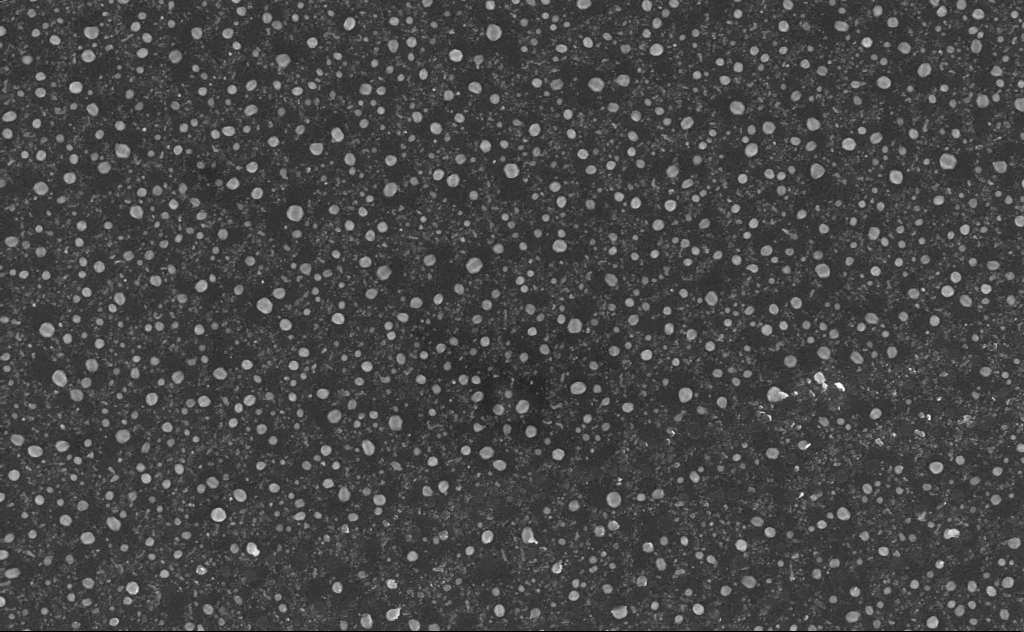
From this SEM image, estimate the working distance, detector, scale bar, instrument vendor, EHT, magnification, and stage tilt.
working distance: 4 mm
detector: InLens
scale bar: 1000 nm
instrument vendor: Zeiss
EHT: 5 kV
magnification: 40 K X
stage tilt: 0°